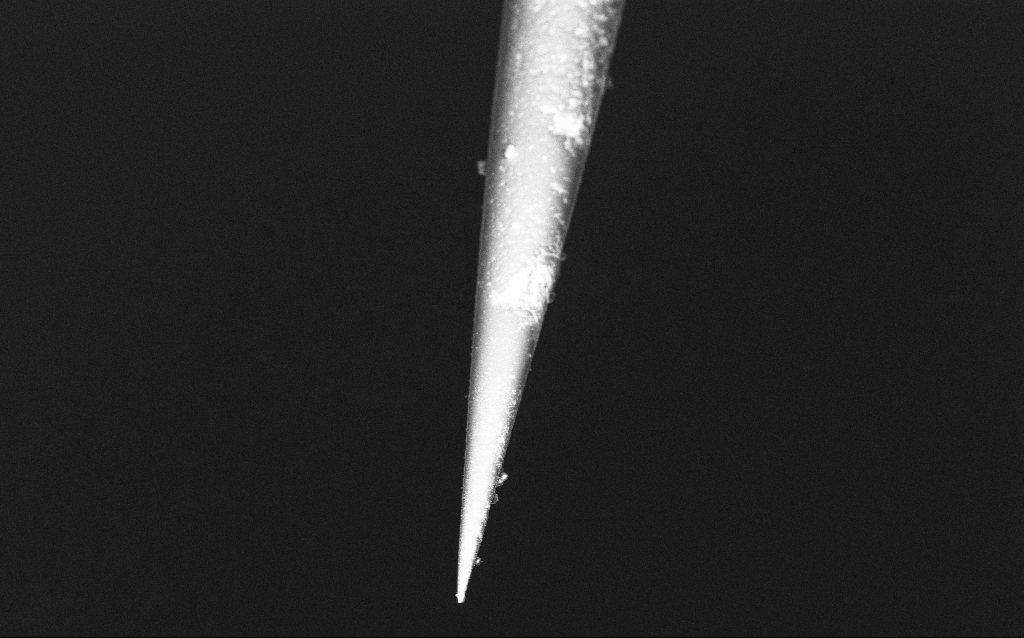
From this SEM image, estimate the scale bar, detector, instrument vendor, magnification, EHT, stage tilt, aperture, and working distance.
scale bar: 10000 nm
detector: InLens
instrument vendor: Zeiss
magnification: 2.5 K X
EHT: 5 kV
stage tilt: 43.9°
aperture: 30 µm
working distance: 5.8 mm